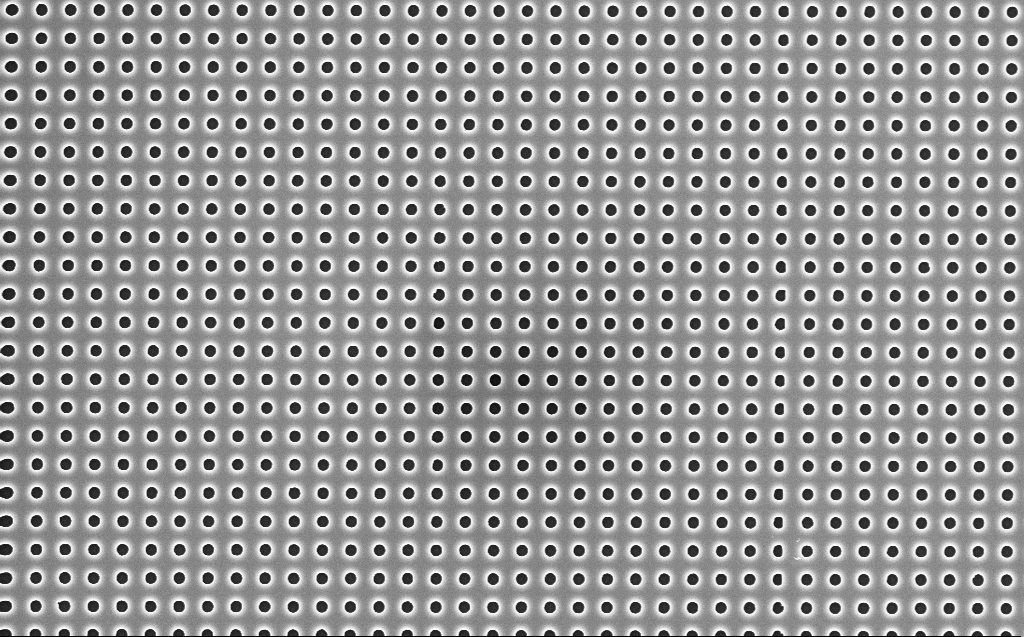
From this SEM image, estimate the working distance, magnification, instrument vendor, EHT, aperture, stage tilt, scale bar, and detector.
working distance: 7 mm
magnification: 10 K X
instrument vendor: Zeiss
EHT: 5 kV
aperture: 30 µm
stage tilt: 0°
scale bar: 2000 nm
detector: InLens